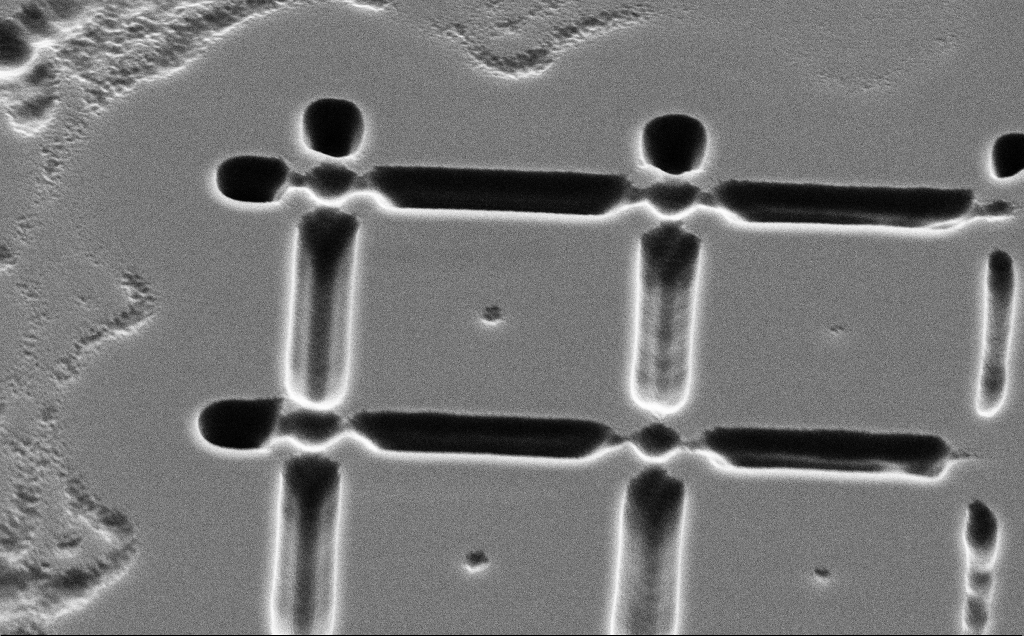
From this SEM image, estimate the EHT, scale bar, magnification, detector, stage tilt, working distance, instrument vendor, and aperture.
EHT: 5 kV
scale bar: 2000 nm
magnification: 12.47 K X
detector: SE2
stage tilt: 45°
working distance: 11 mm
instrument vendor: Zeiss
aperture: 30 µm